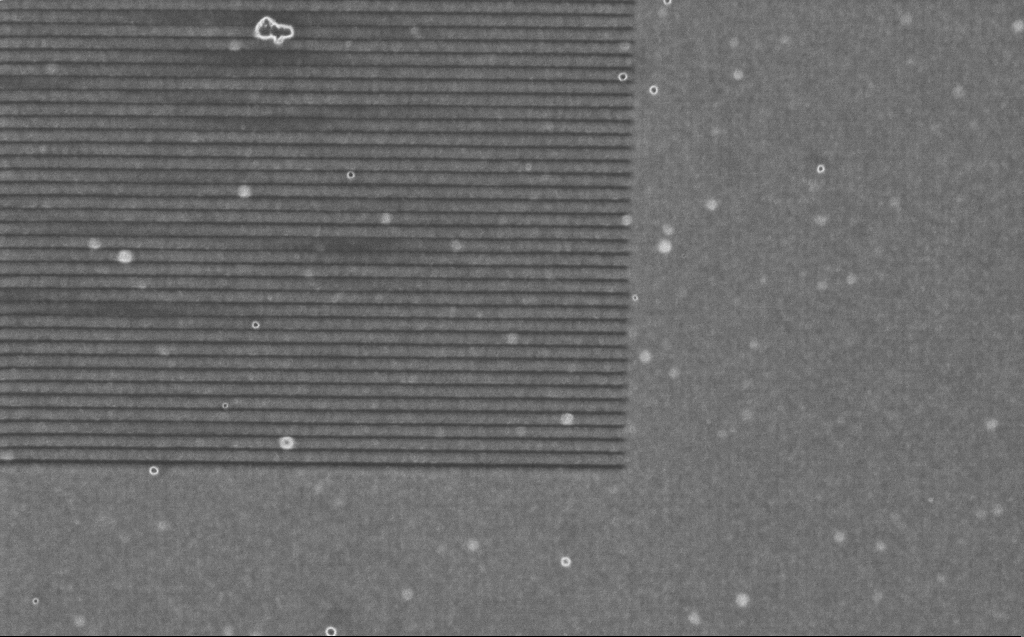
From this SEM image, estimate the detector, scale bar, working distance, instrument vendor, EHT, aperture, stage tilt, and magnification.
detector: InLens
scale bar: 2000 nm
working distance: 4 mm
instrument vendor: Zeiss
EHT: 1 kV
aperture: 30 µm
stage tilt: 0°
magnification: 24.59 K X